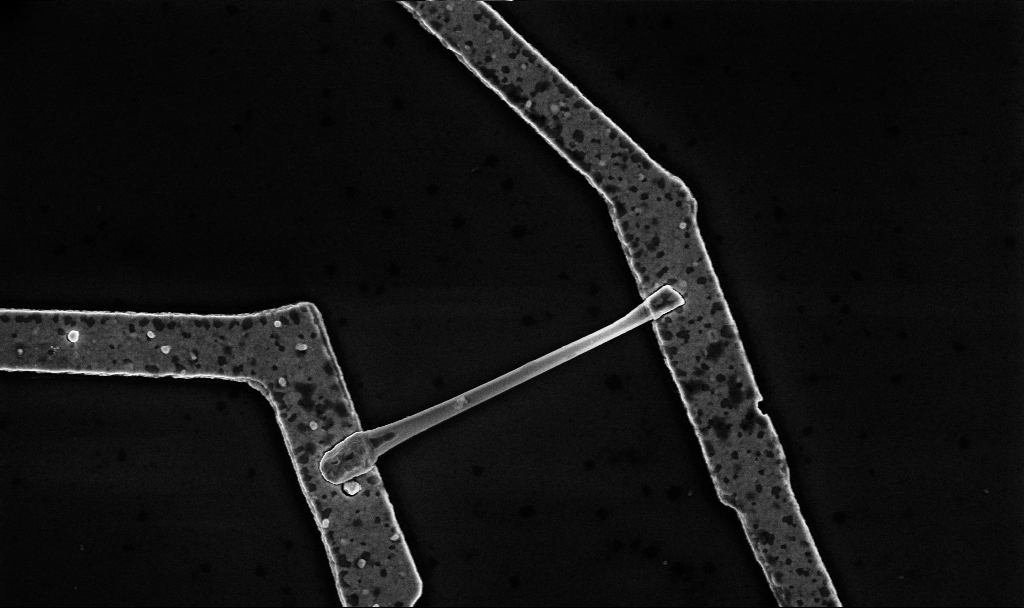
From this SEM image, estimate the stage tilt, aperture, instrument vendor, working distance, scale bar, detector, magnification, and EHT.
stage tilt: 0°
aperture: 30 µm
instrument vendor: Zeiss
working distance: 8.7 mm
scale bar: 1000 nm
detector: InLens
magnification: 30 K X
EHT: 5 kV